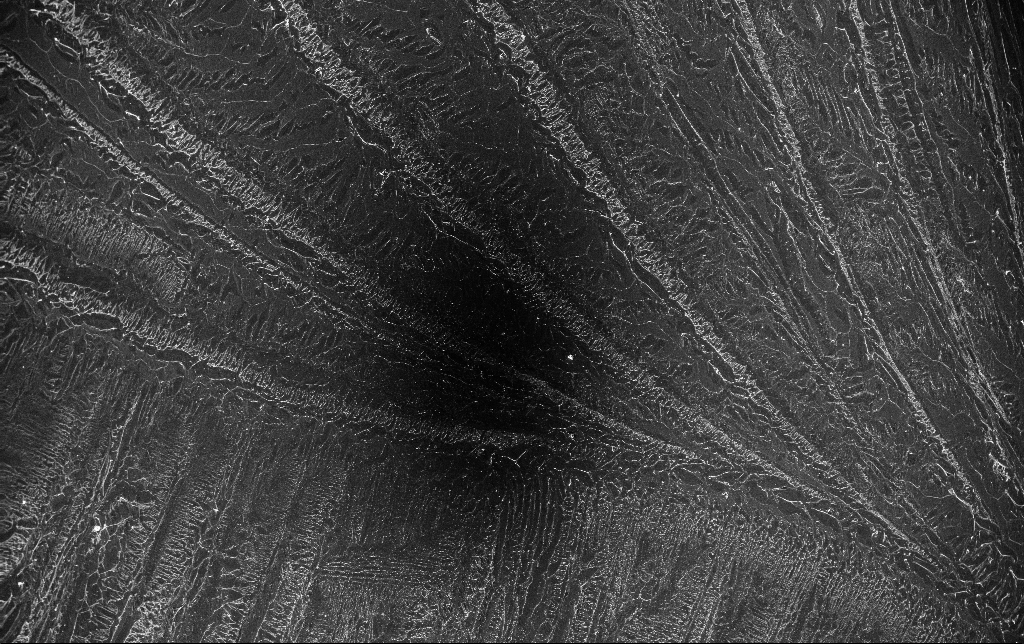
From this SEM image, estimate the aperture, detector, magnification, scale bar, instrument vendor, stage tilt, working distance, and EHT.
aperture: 30 µm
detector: InLens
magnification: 0.153 K X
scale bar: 100000 nm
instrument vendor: Zeiss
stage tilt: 0°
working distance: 3.2 mm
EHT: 10 kV